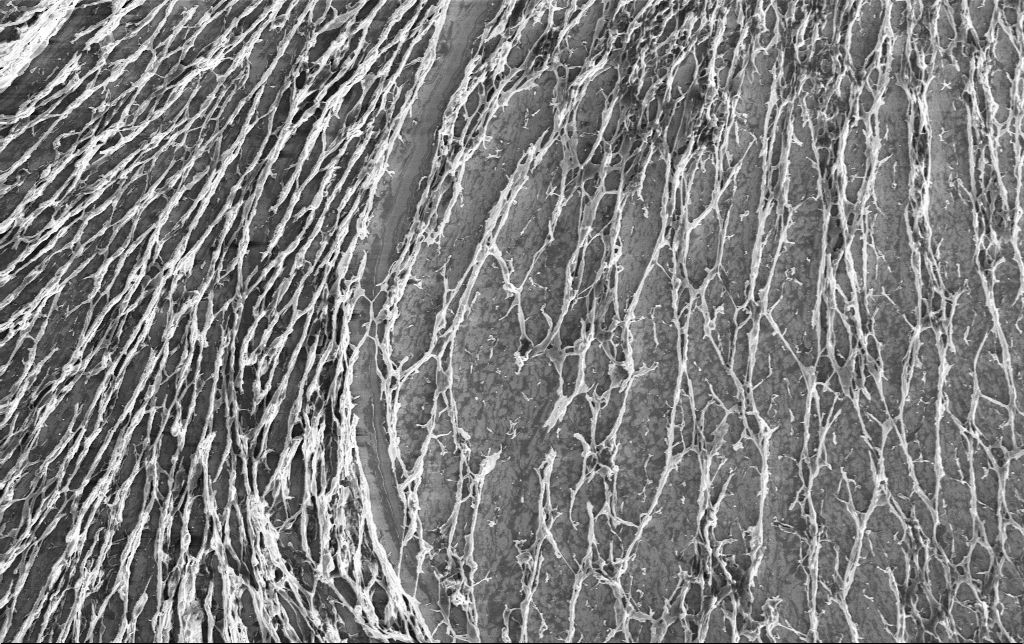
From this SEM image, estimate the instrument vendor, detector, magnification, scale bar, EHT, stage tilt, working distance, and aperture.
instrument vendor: Zeiss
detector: InLens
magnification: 2.97 K X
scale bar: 10000 nm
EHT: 3 kV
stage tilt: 0°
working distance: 3.2 mm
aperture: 30 µm